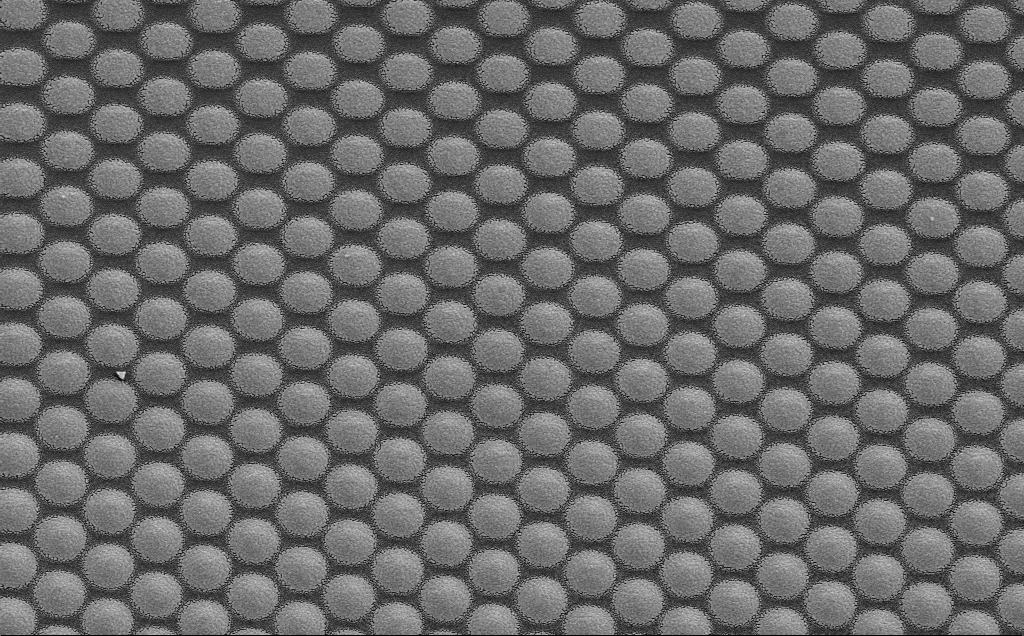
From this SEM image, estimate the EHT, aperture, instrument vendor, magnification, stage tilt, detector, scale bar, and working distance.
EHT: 5 kV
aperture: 30 µm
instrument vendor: Zeiss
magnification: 6.72 K X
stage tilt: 0°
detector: SE2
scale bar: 10000 nm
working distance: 20 mm